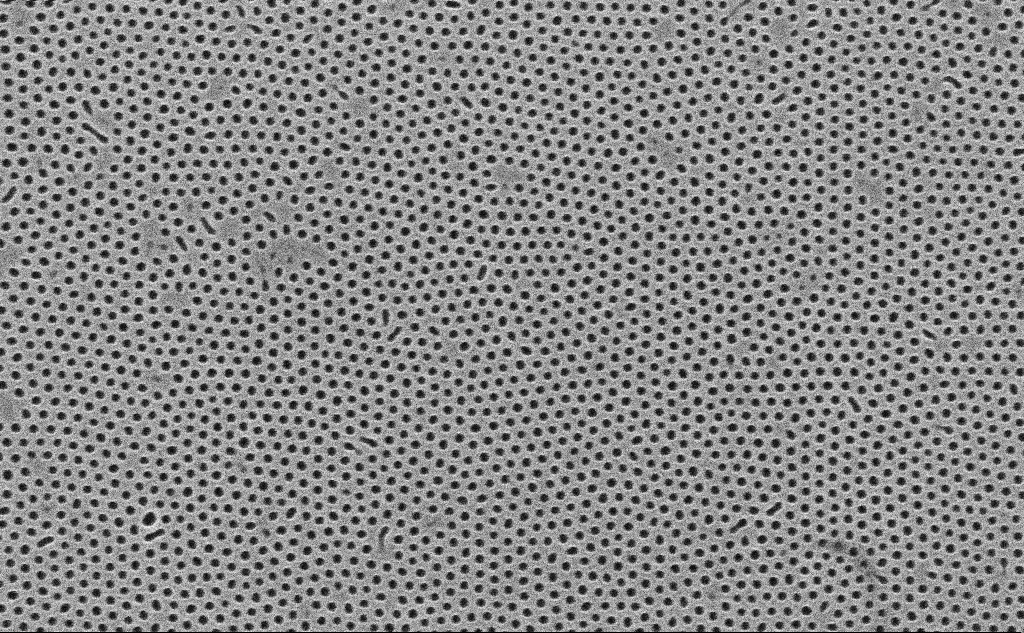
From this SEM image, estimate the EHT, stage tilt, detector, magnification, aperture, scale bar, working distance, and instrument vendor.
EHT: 10 kV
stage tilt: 0°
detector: InLens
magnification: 132.27 K X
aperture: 30 µm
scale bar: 200 nm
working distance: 4.2 mm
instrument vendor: Zeiss